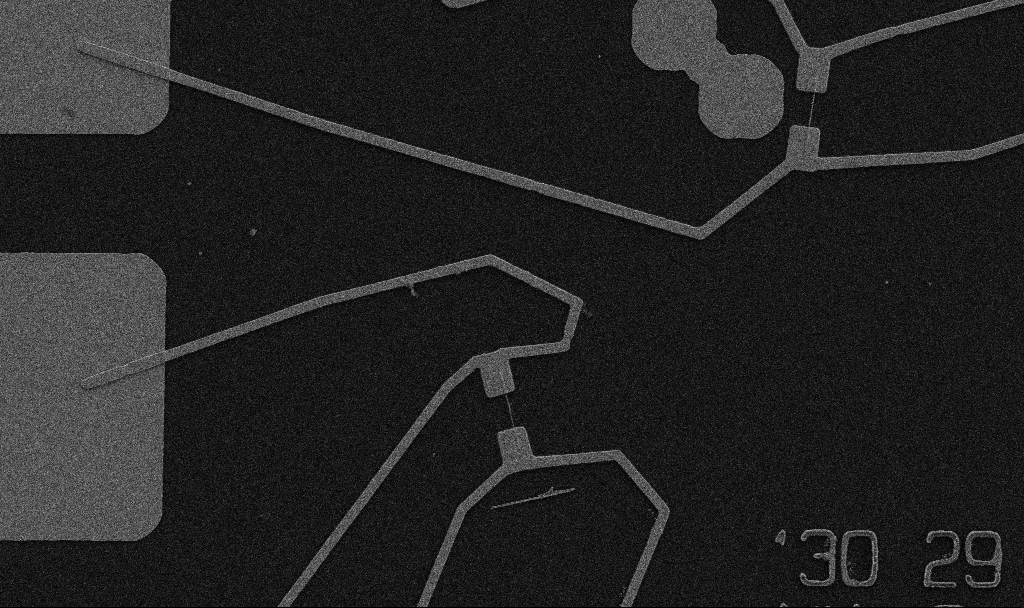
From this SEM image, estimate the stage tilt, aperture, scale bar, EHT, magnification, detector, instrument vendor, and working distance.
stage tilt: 0°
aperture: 30 µm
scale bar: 10000 nm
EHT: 5 kV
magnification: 5 K X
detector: SE2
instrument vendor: Zeiss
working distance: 10.7 mm